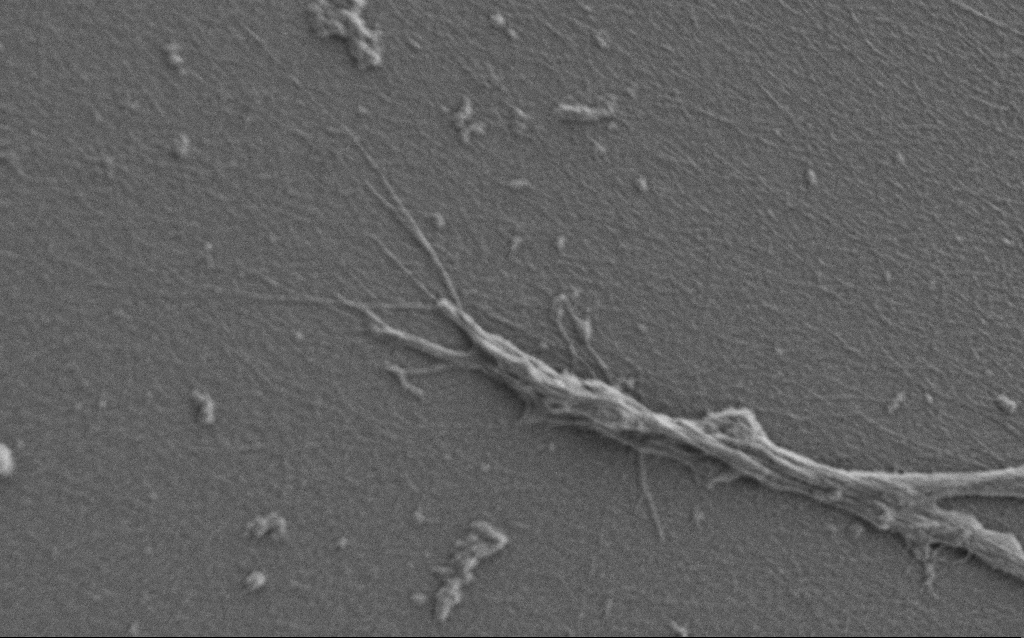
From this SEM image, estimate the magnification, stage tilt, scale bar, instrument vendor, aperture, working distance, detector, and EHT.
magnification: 10 K X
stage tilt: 0°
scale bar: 2000 nm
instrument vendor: Zeiss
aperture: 30 µm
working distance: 7 mm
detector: SE2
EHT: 0.9 kV